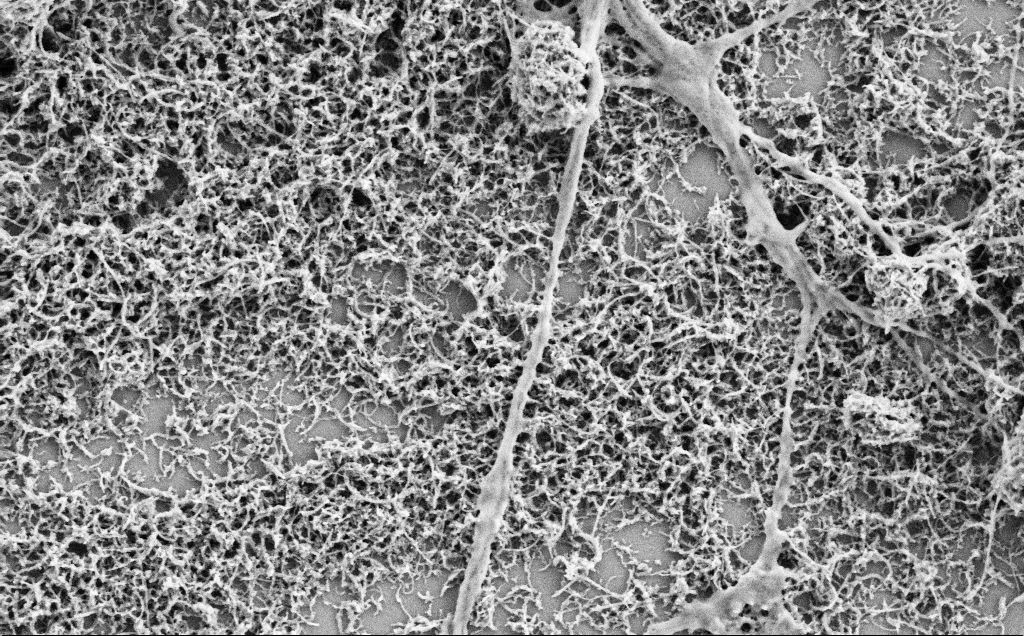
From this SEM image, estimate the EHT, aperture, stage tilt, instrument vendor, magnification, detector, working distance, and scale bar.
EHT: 2 kV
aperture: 30 µm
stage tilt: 0°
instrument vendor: Zeiss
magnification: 25 K X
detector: SE2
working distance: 7.1 mm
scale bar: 1000 nm